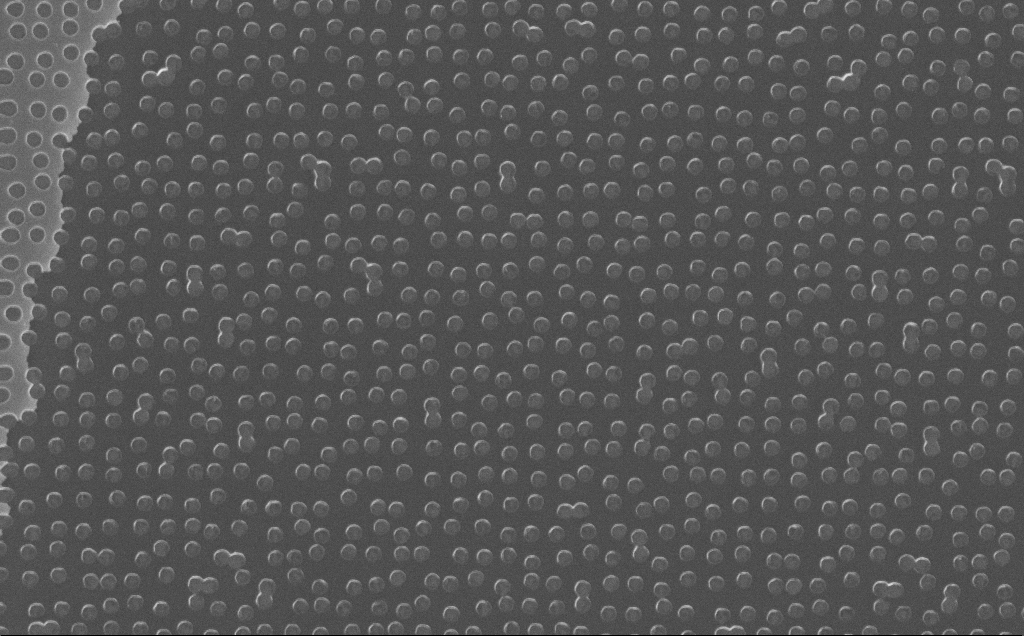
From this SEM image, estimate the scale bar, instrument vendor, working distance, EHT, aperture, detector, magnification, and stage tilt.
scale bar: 2000 nm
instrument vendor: Zeiss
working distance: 7 mm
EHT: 10 kV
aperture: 30 µm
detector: InLens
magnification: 24.27 K X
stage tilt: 0°